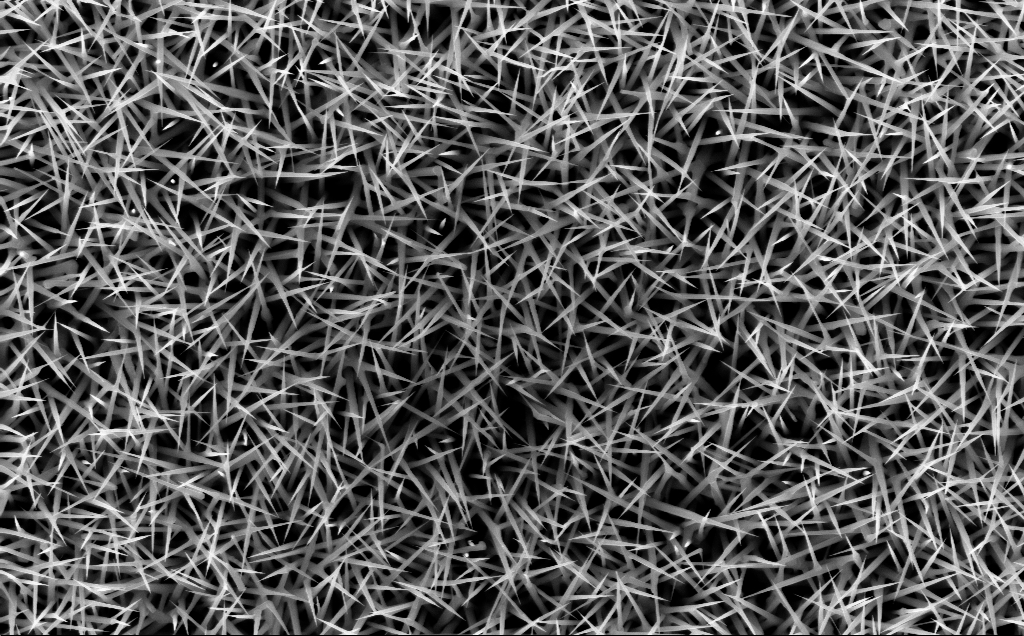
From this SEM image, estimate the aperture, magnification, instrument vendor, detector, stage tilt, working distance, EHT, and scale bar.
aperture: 30 µm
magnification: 20 K X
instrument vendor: Zeiss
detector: InLens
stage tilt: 0°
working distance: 7 mm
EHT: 10 kV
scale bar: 1000 nm